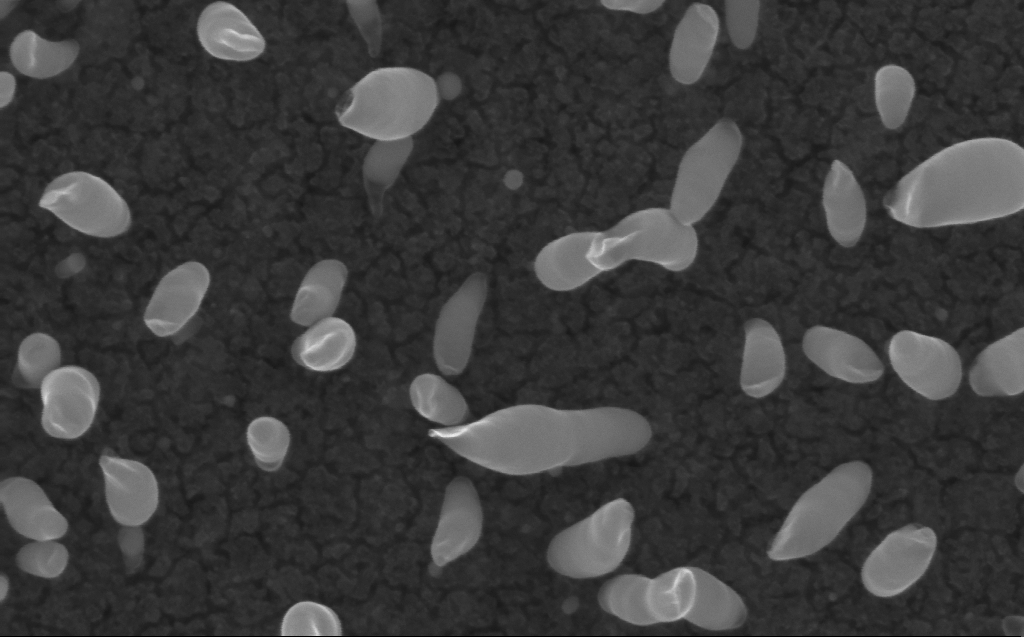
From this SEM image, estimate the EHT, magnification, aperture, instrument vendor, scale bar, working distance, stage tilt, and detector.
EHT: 10 kV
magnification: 200 K X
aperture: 30 µm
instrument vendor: Zeiss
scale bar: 100 nm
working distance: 3 mm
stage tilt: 0°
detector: InLens